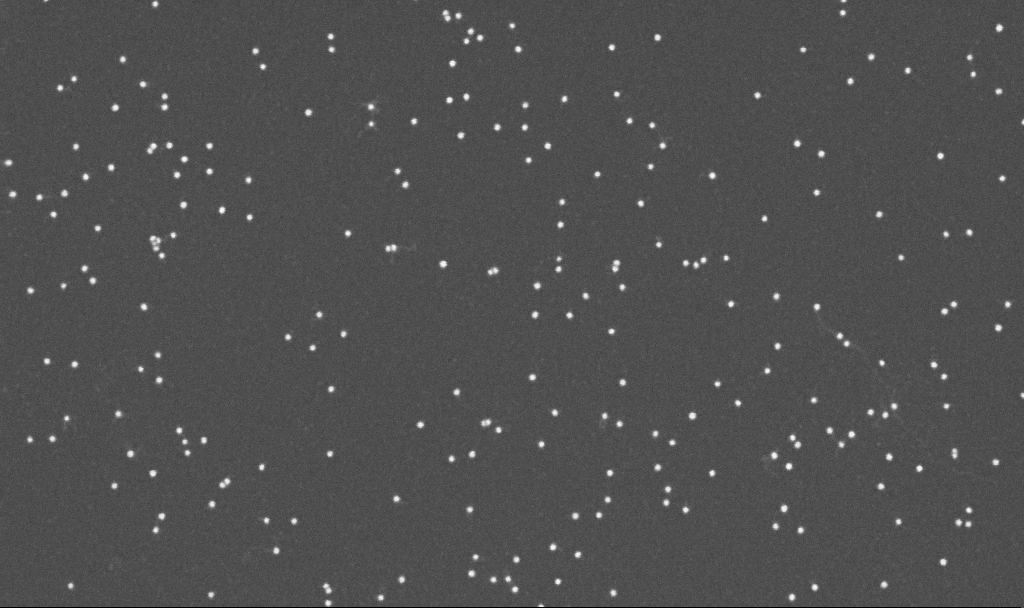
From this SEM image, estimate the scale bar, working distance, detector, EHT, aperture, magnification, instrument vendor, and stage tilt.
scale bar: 200 nm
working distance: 3.3 mm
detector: InLens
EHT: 10 kV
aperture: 30 µm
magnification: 200 K X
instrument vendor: Zeiss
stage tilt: -0°